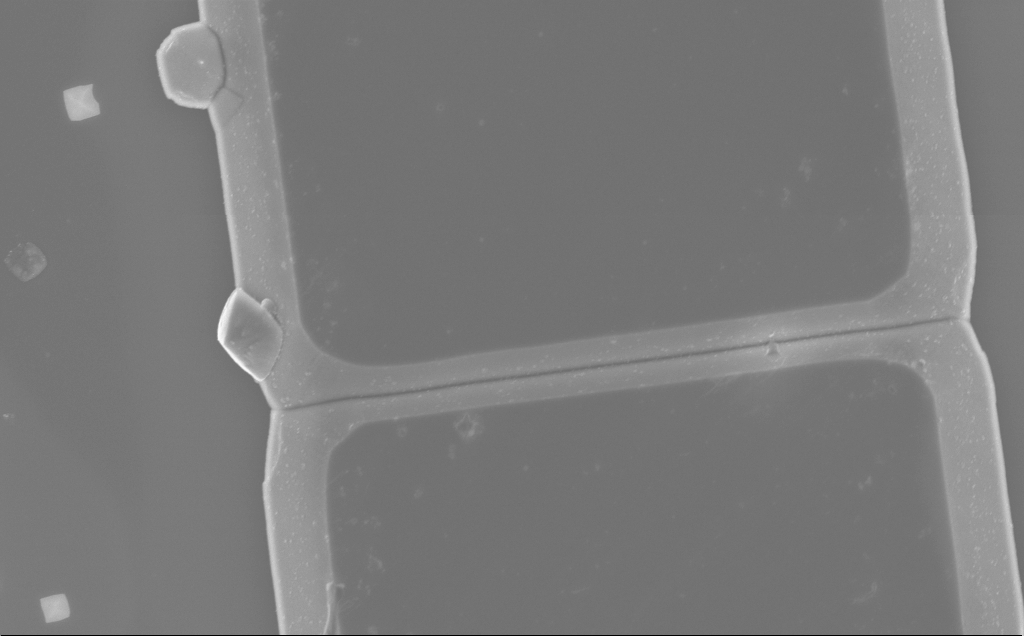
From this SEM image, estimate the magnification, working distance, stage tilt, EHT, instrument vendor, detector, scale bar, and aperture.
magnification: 11.35 K X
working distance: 10 mm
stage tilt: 0°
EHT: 5 kV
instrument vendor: Zeiss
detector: InLens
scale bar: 2000 nm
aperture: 30 µm